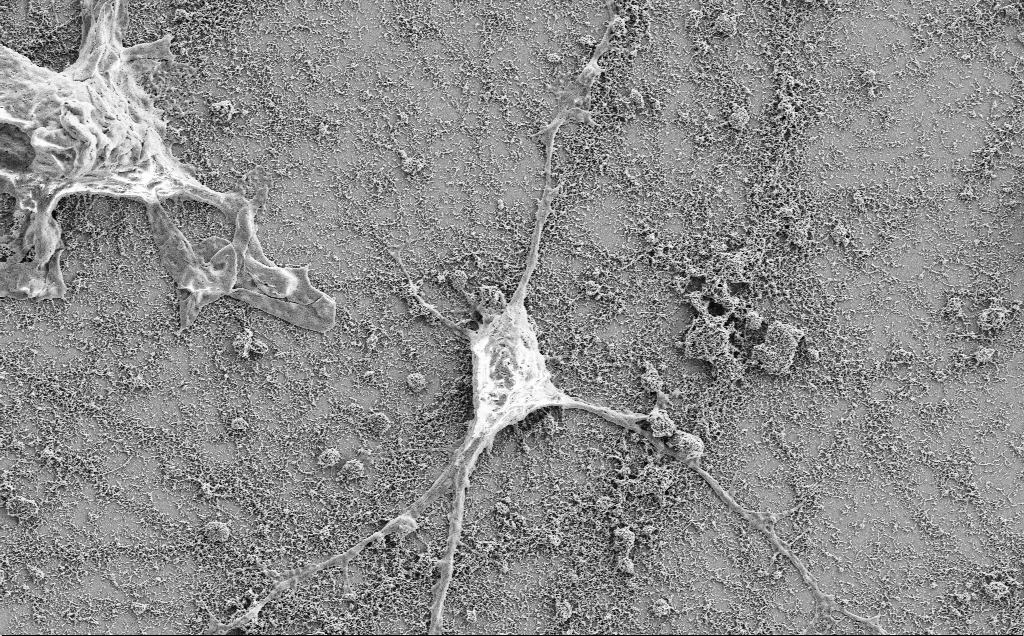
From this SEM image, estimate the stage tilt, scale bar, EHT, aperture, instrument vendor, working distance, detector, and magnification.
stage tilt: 0°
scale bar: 10000 nm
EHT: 2 kV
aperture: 30 µm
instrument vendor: Zeiss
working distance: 7.1 mm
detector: SE2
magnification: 5 K X